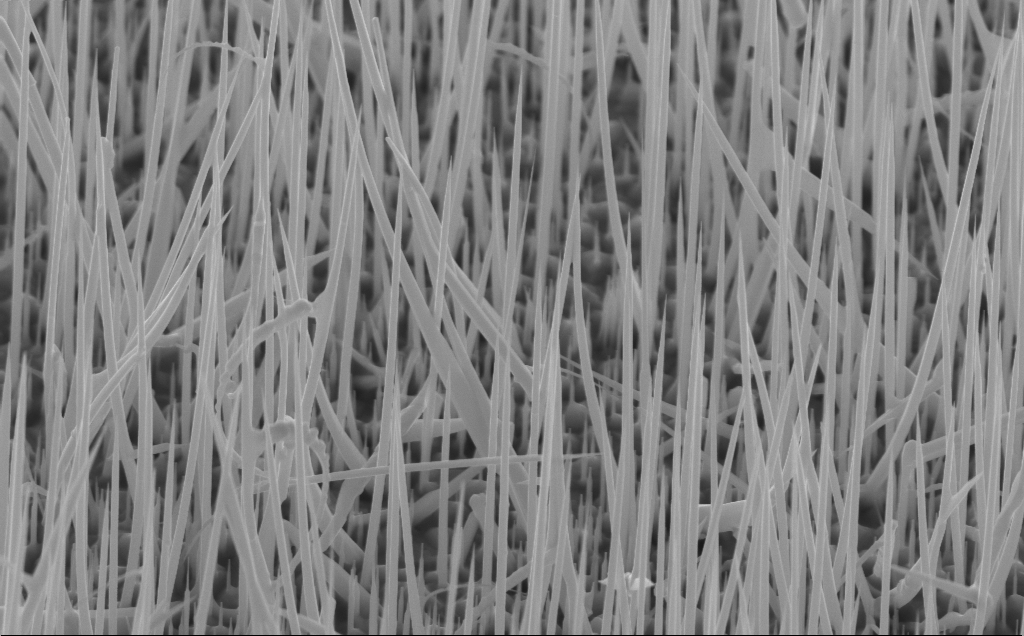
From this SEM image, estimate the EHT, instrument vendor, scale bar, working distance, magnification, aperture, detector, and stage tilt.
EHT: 10 kV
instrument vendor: Zeiss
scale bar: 1000 nm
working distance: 4 mm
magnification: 27.71 K X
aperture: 30 µm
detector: InLens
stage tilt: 45°